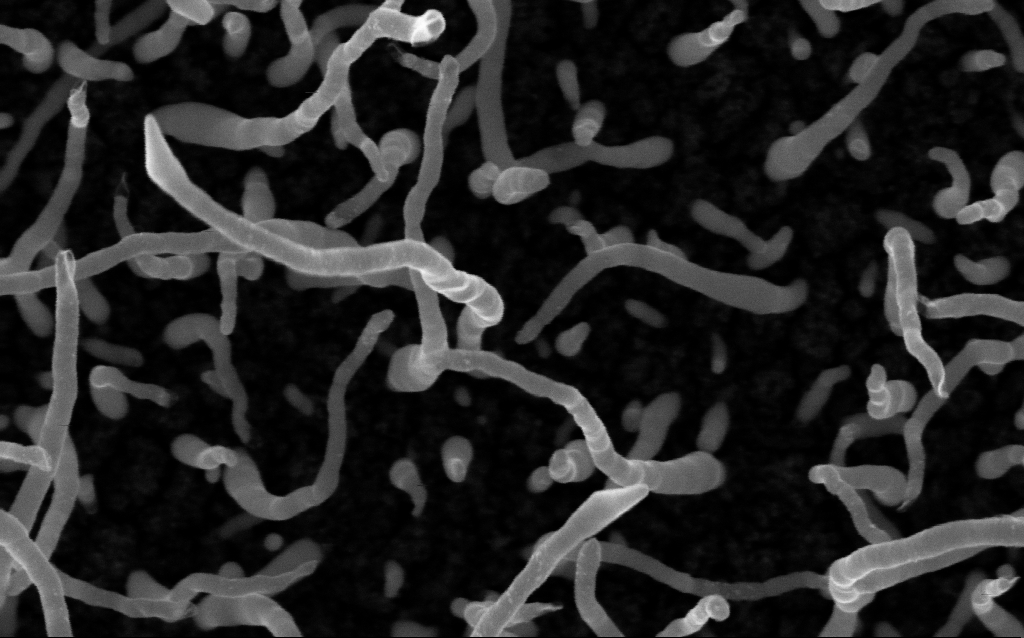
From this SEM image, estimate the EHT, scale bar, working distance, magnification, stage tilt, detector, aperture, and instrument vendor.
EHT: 5 kV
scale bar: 100 nm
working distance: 2 mm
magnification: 200 K X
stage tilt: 0°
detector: InLens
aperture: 30 µm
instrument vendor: Zeiss